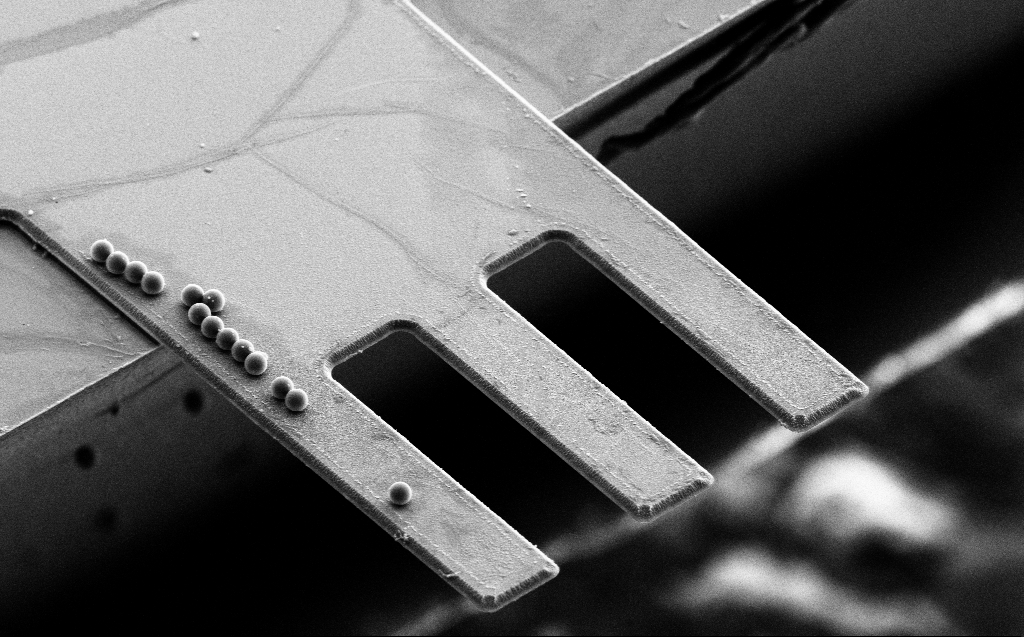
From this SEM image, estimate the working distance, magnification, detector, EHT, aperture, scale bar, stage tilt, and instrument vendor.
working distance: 11 mm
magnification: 1.85 K X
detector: SE2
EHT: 10 kV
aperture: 30 µm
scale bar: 10000 nm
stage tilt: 45.4°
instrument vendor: Zeiss